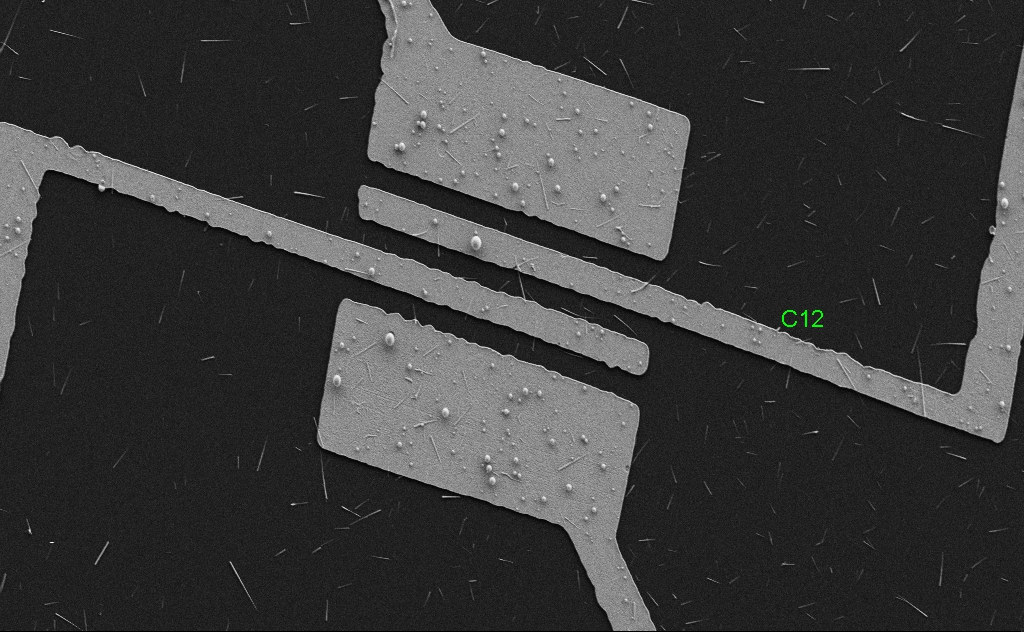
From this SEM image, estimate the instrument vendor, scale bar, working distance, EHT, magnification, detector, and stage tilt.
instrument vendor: Zeiss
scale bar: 10000 nm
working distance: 5 mm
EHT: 5 kV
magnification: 4 K X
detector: SE2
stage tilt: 0°